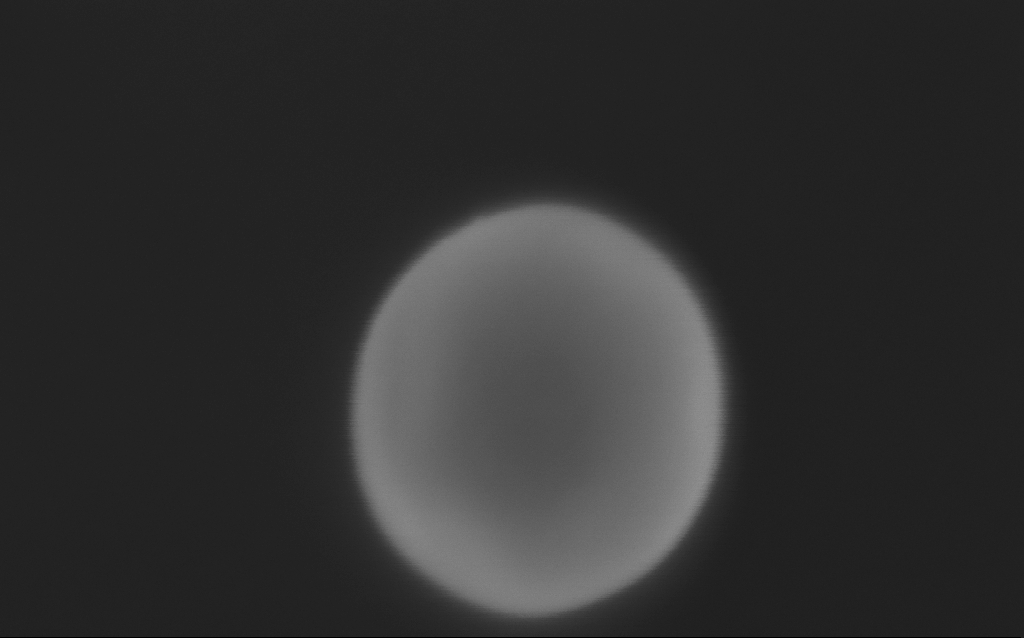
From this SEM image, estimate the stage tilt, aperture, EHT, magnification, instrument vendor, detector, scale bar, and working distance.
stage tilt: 0°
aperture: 30 µm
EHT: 5 kV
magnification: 651.83 K X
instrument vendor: Zeiss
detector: InLens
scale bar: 100 nm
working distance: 3 mm